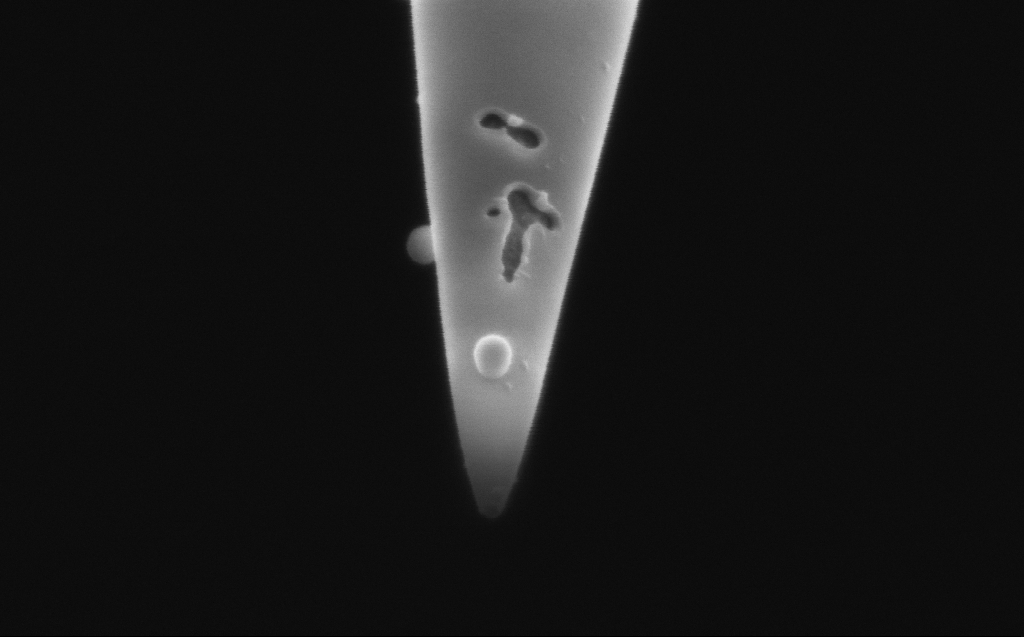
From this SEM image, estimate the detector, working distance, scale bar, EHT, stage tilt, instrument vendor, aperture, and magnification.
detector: InLens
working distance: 3 mm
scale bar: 200 nm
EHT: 2 kV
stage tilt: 45.1°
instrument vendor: Zeiss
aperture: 20 µm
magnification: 131.69 K X